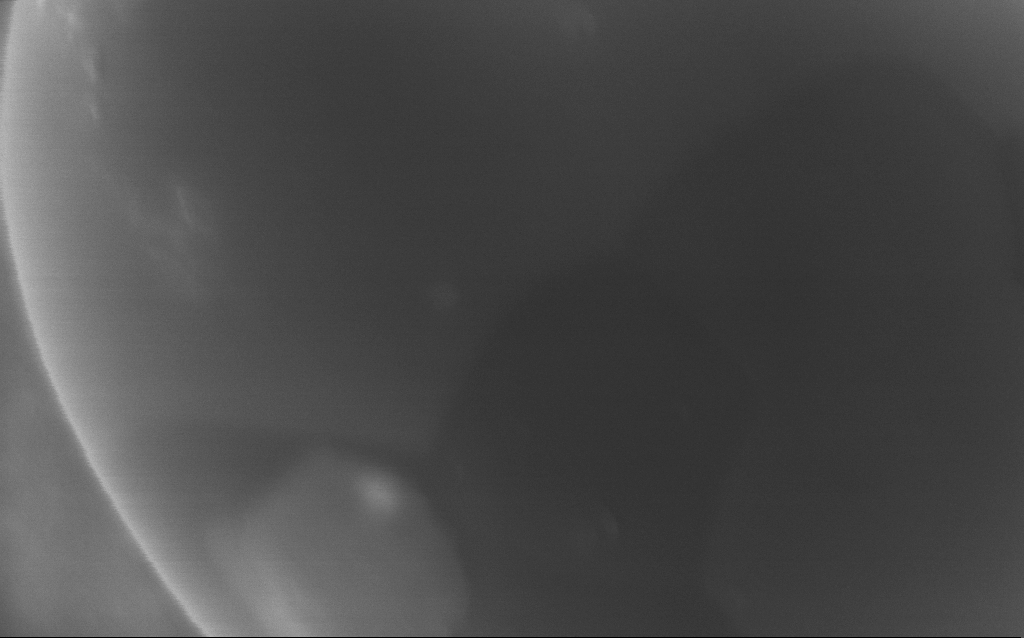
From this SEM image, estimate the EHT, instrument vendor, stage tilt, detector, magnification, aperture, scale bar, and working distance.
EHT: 5 kV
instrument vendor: Zeiss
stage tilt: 0°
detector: InLens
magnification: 270 K X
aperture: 30 µm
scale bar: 100 nm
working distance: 4 mm